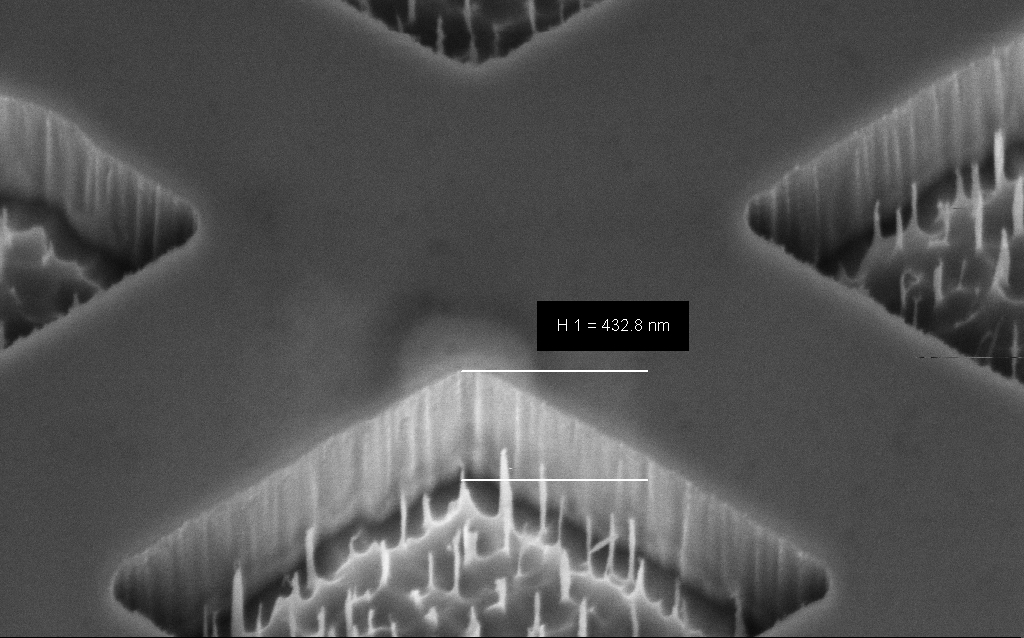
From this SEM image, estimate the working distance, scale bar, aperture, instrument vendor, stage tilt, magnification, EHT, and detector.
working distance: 8 mm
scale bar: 200 nm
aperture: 30 µm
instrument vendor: Zeiss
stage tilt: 45°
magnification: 92.48 K X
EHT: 3 kV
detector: InLens